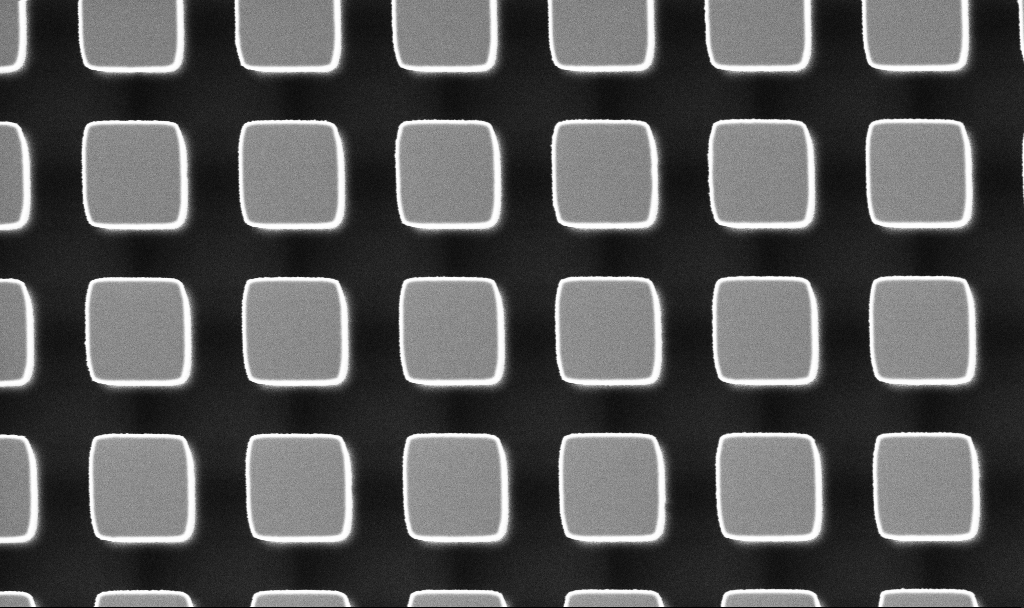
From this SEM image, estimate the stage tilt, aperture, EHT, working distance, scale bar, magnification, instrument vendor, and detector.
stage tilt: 0°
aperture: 30 µm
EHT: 3 kV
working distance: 3.2 mm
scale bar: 2000 nm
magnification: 9.69 K X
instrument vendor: Zeiss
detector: InLens